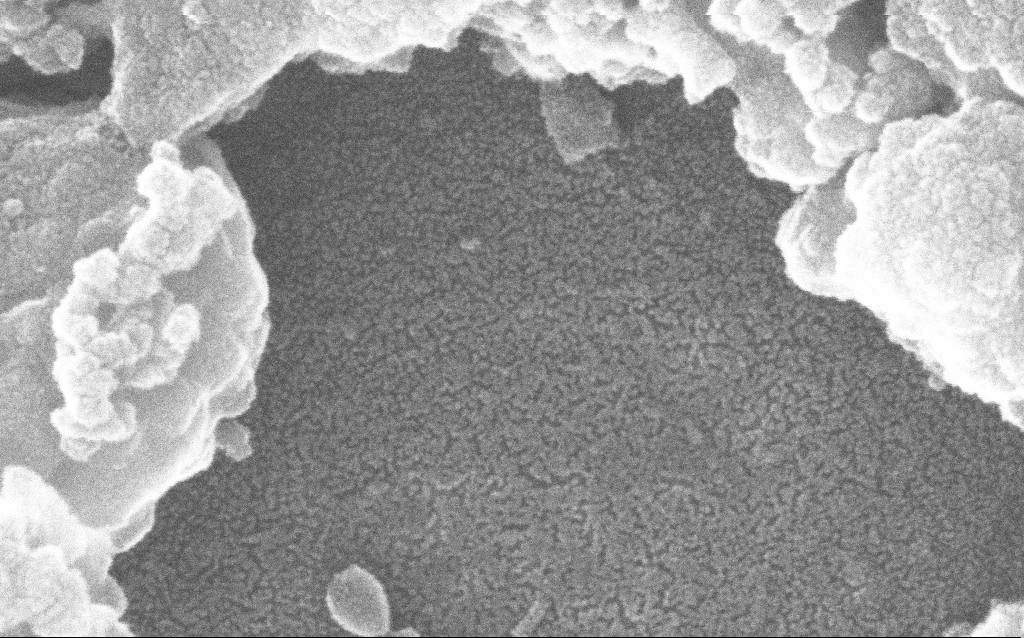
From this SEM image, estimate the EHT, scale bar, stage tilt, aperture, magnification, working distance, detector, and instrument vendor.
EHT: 20 kV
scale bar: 100 nm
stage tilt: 0°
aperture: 30 µm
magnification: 500 K X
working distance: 1.7 mm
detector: InLens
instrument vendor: Zeiss